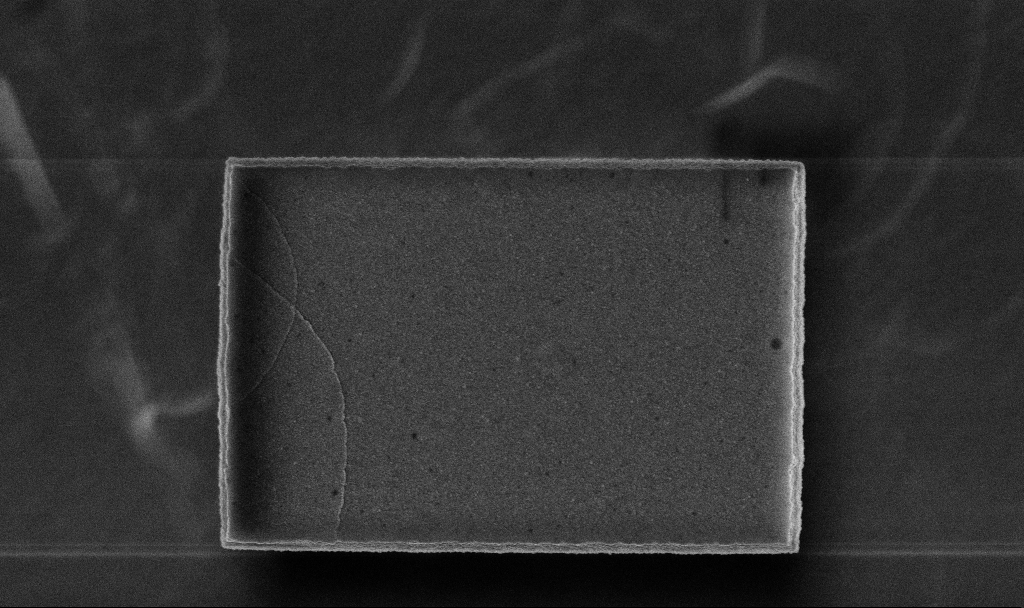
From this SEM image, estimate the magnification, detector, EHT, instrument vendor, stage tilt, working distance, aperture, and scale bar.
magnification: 47.83 K X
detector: InLens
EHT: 5 kV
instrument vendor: Zeiss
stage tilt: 0°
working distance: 3.2 mm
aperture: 30 µm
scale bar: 1000 nm